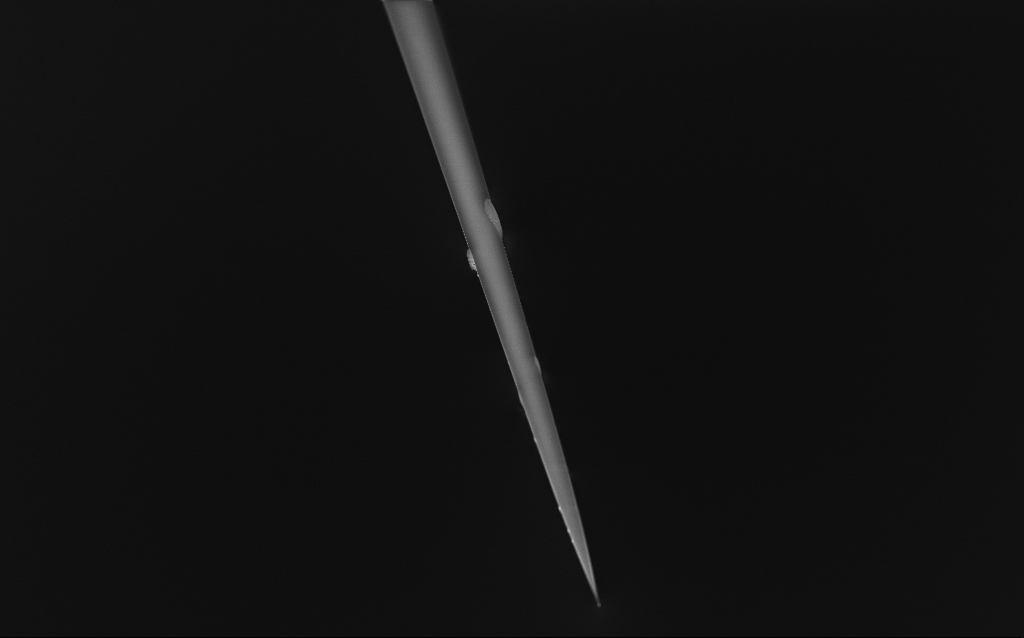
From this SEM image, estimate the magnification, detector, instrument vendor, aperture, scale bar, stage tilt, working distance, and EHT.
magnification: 0.5 K X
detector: InLens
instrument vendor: Zeiss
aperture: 30 µm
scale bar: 100000 nm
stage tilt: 45°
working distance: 6 mm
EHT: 1 kV